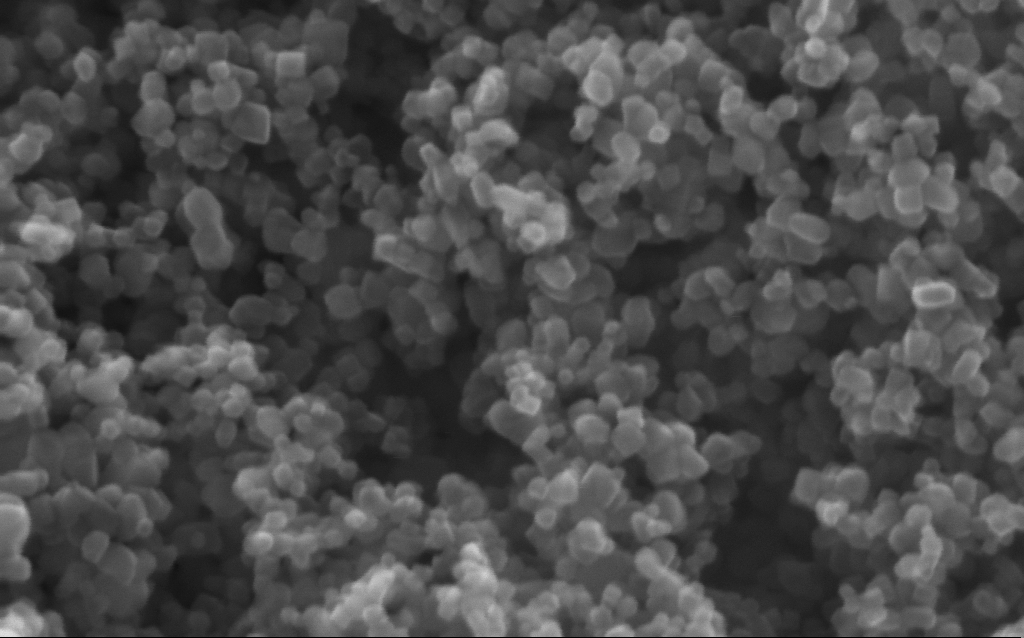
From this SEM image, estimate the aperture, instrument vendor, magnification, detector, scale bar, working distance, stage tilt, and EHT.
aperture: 30 µm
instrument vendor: Zeiss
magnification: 416 K X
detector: InLens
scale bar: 100 nm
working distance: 2.6 mm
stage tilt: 0°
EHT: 10 kV